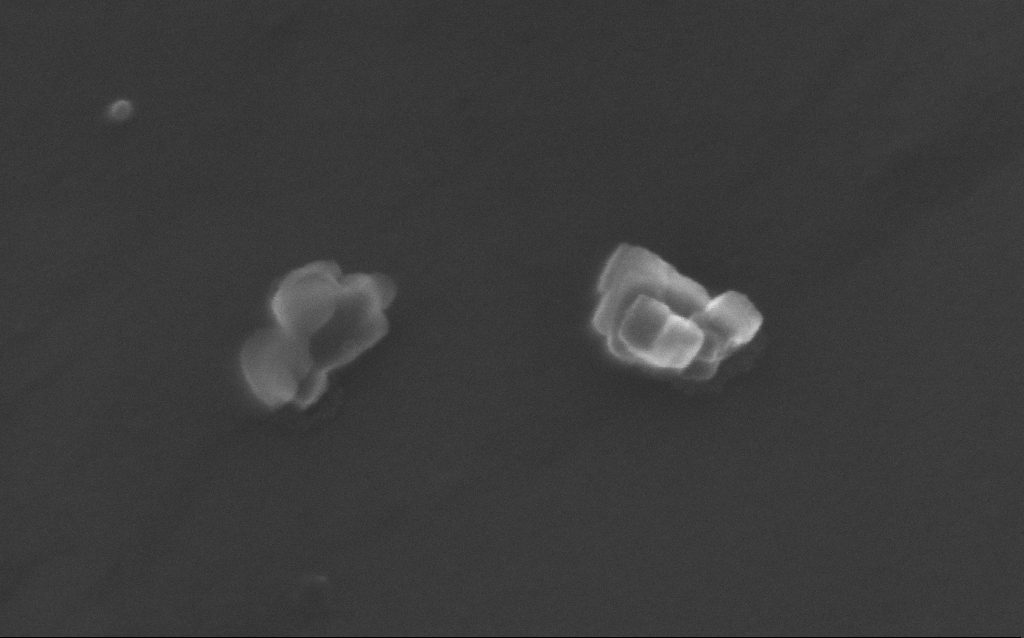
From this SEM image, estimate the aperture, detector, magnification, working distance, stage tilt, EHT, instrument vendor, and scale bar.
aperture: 30 µm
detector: InLens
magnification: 255.23 K X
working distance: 4 mm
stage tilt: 20°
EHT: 10 kV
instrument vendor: Zeiss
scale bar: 200 nm